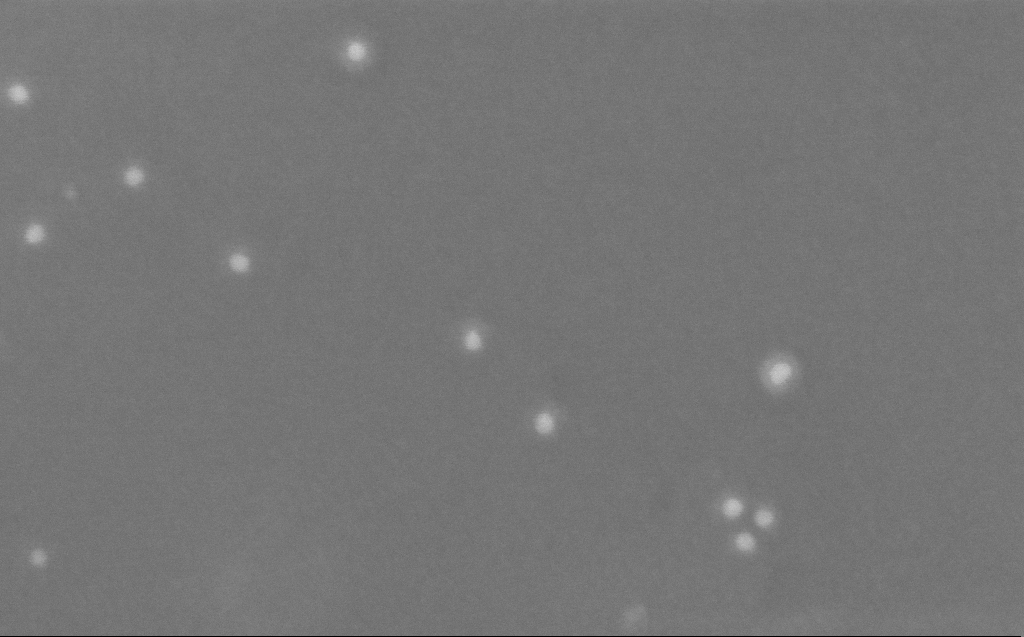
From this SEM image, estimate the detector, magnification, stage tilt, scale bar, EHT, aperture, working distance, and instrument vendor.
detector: InLens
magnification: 444.07 K X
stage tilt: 0°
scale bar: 100 nm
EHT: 10 kV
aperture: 30 µm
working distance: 3 mm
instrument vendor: Zeiss